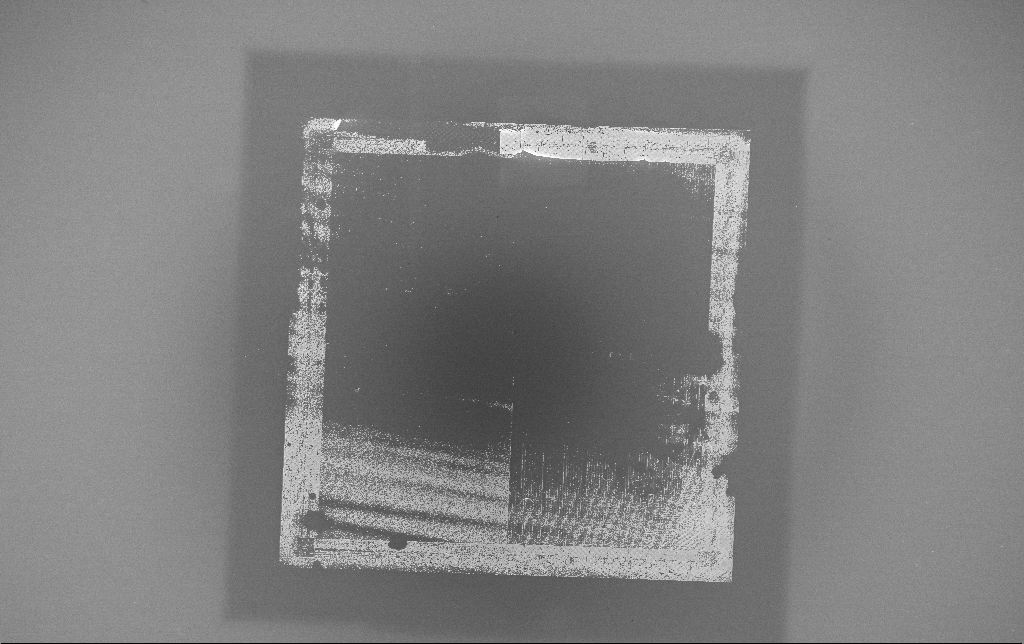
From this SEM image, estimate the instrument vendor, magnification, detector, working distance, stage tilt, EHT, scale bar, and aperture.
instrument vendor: Zeiss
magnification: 0.167 K X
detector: InLens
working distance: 4.7 mm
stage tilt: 0°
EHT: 5 kV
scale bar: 200000 nm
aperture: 30 µm